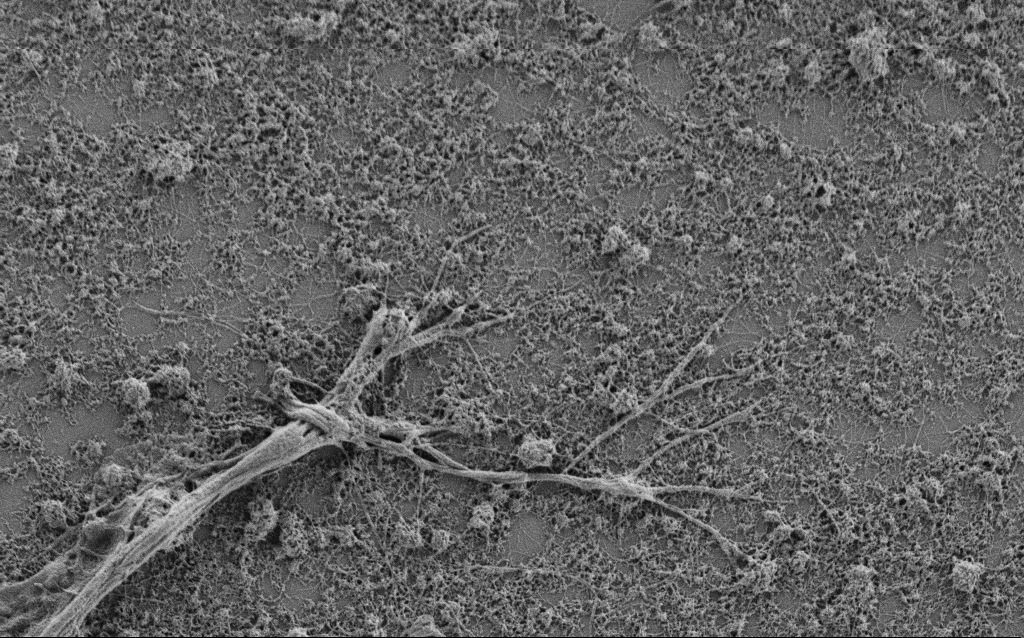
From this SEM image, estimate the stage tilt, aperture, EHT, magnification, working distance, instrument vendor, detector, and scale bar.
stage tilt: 0°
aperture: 30 µm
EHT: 0.9 kV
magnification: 7.5 K X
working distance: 4 mm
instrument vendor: Zeiss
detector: SE2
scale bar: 2000 nm